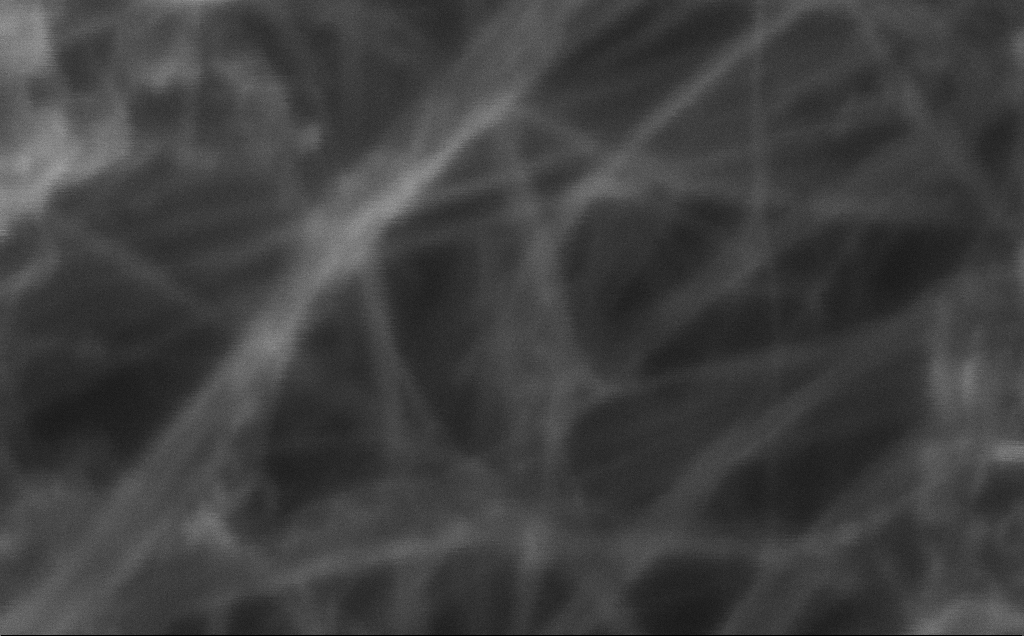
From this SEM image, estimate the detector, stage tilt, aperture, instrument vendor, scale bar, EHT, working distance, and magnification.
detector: InLens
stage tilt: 0°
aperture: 30 µm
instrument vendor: Zeiss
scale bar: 20 nm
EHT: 10 kV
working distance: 3 mm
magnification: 926.07 K X